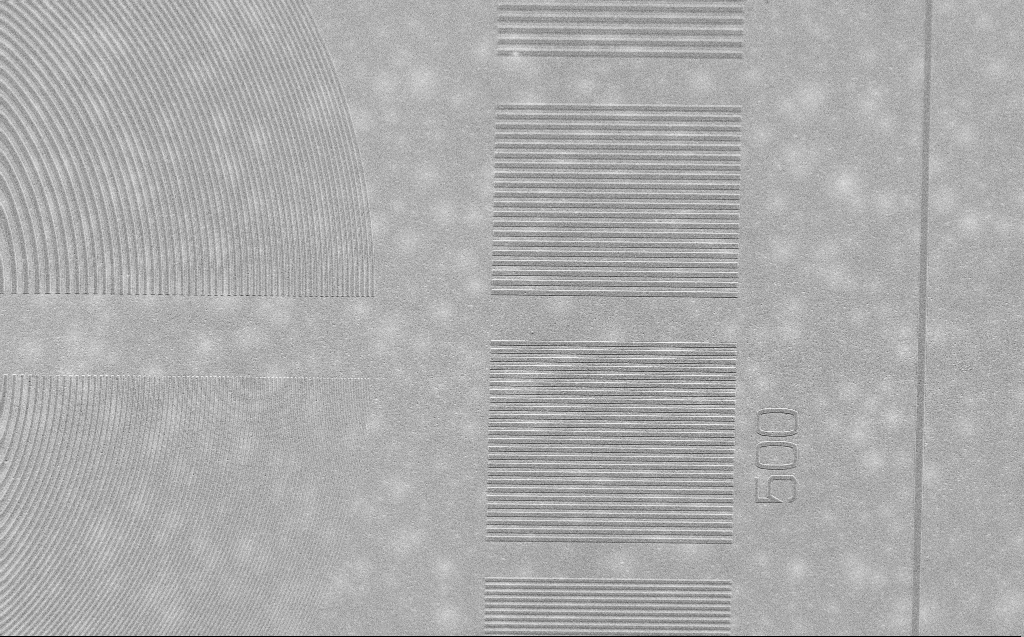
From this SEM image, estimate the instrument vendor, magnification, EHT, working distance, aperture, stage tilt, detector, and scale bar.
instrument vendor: Zeiss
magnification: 3.06 K X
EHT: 2.5 kV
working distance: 4 mm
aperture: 30 µm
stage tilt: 30°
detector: SE2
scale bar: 10000 nm